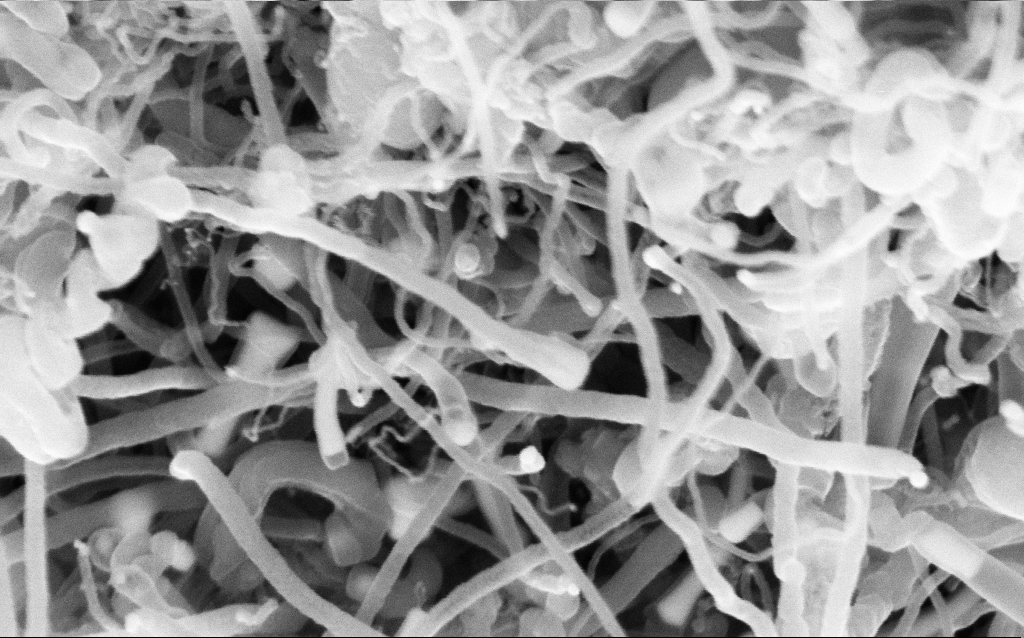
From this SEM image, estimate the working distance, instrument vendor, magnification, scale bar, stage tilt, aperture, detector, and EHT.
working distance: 6.6 mm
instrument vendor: Zeiss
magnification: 162.1 K X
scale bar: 200 nm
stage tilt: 45°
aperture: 30 µm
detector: InLens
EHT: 5 kV